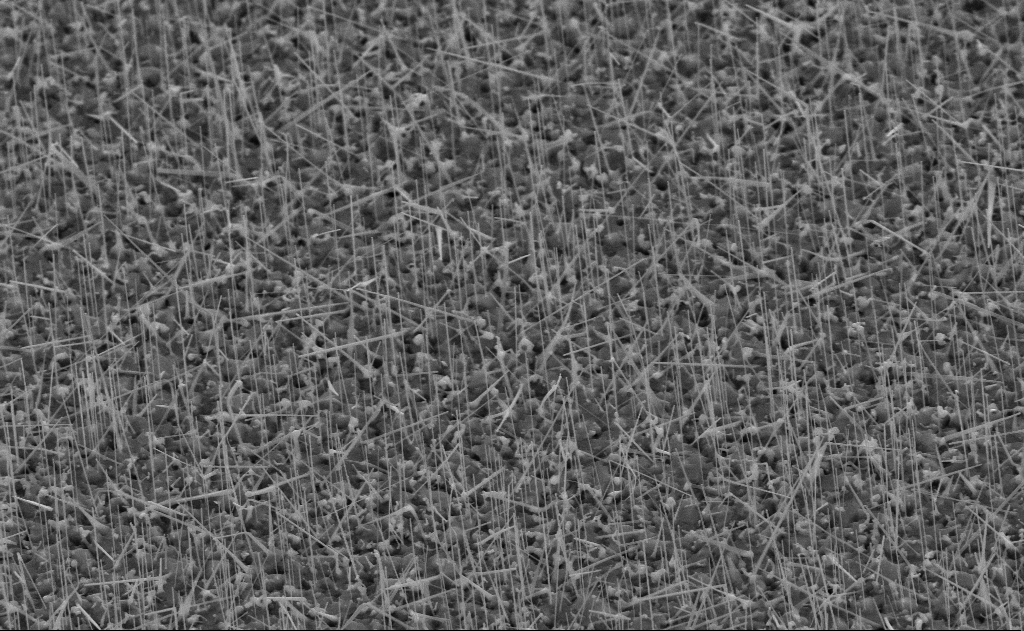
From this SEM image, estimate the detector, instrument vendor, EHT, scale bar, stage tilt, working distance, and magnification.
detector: SE2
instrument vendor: Zeiss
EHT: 10 kV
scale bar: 1000 nm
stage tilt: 45°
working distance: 11 mm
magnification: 20 K X